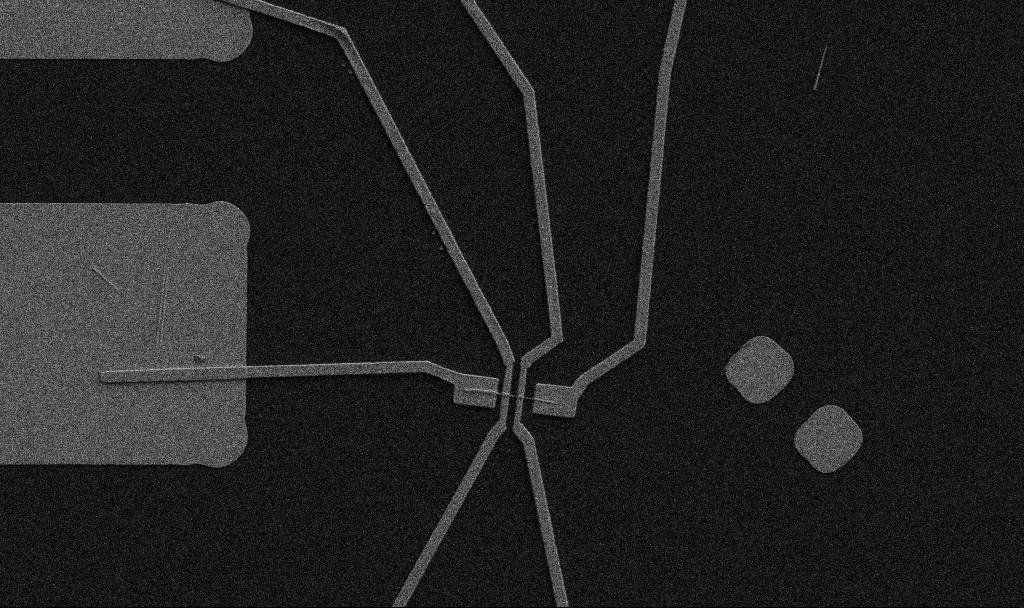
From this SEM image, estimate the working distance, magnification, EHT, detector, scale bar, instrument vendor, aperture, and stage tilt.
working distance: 10.7 mm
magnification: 5 K X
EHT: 5 kV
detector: SE2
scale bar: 10000 nm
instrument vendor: Zeiss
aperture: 30 µm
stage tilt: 0°